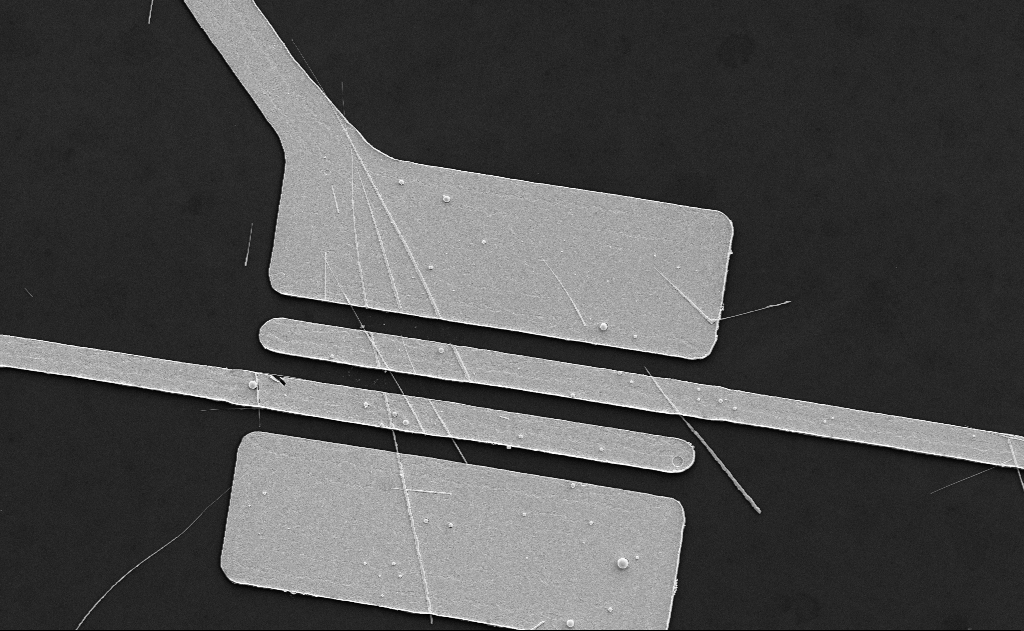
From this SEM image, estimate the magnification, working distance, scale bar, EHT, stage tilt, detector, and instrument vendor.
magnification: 5.43 K X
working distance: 19 mm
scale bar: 10000 nm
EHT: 5 kV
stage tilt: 0°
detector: SE2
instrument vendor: Zeiss